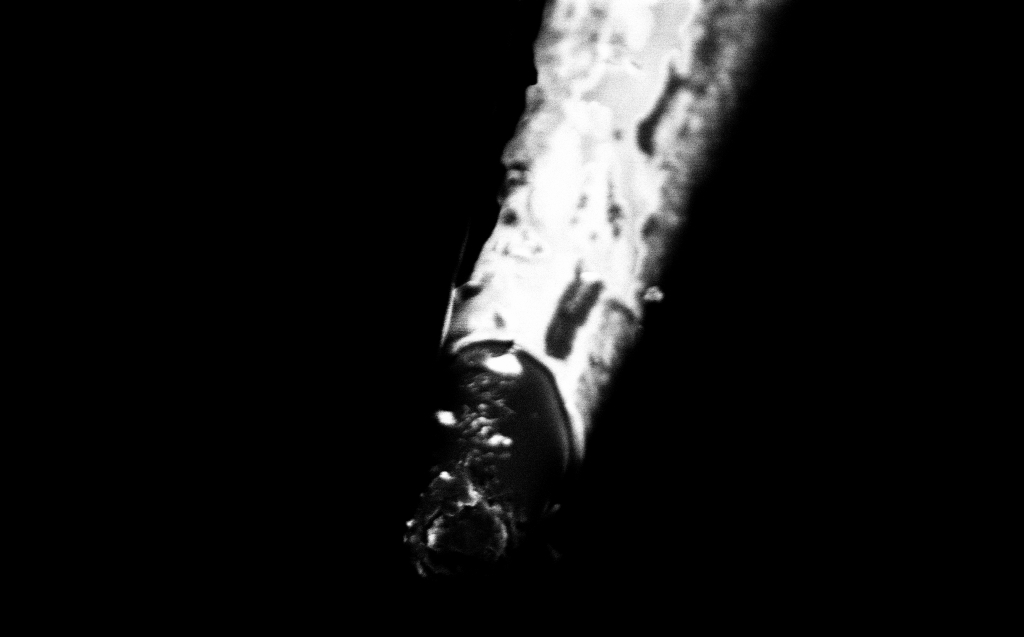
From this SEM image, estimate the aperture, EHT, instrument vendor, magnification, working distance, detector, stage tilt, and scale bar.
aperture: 30 µm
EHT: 2 kV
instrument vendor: Zeiss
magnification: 50 K X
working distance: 5 mm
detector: InLens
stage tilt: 45°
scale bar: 1000 nm